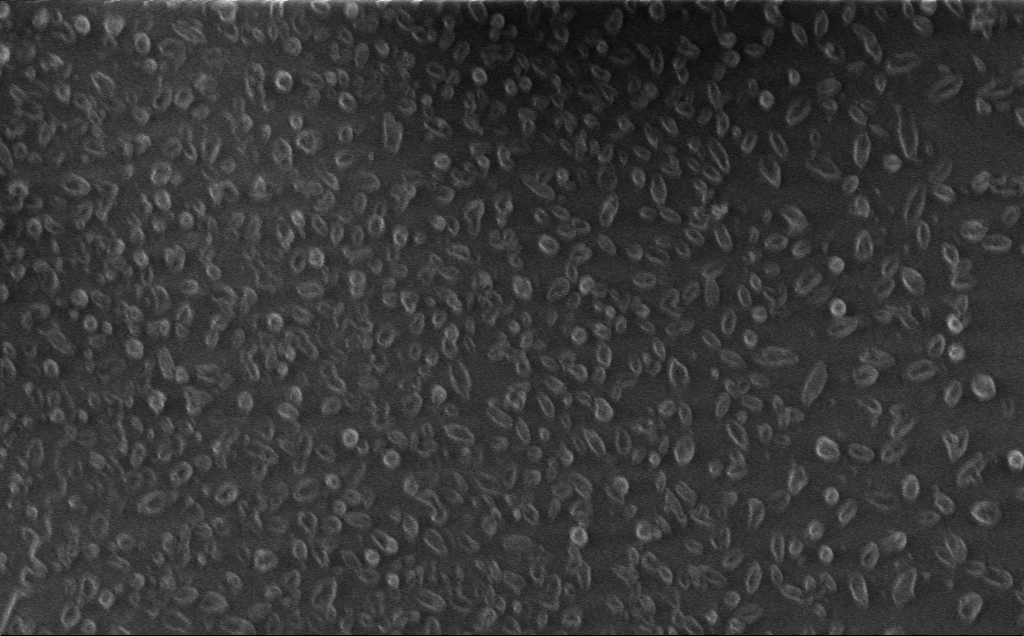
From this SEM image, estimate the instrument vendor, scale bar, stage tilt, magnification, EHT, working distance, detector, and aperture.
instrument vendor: Zeiss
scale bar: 2000 nm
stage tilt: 0°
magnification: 7.42 K X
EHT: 1 kV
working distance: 3 mm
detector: InLens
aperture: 30 µm